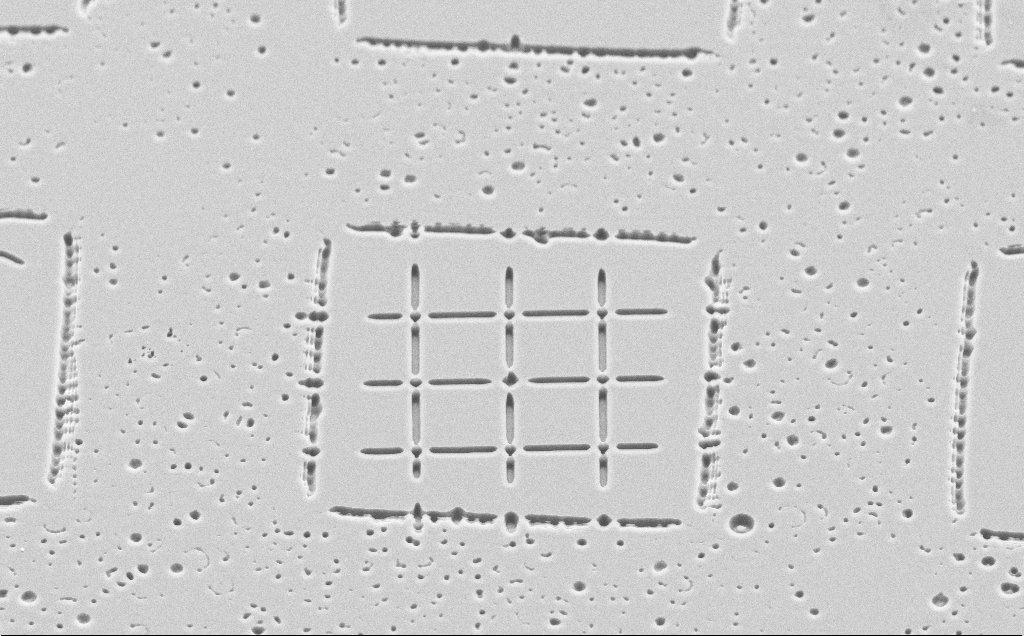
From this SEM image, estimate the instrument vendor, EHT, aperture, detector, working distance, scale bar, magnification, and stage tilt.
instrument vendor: Zeiss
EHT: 10 kV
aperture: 30 µm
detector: SE2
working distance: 6 mm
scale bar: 20000 nm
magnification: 1.7 K X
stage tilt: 45°